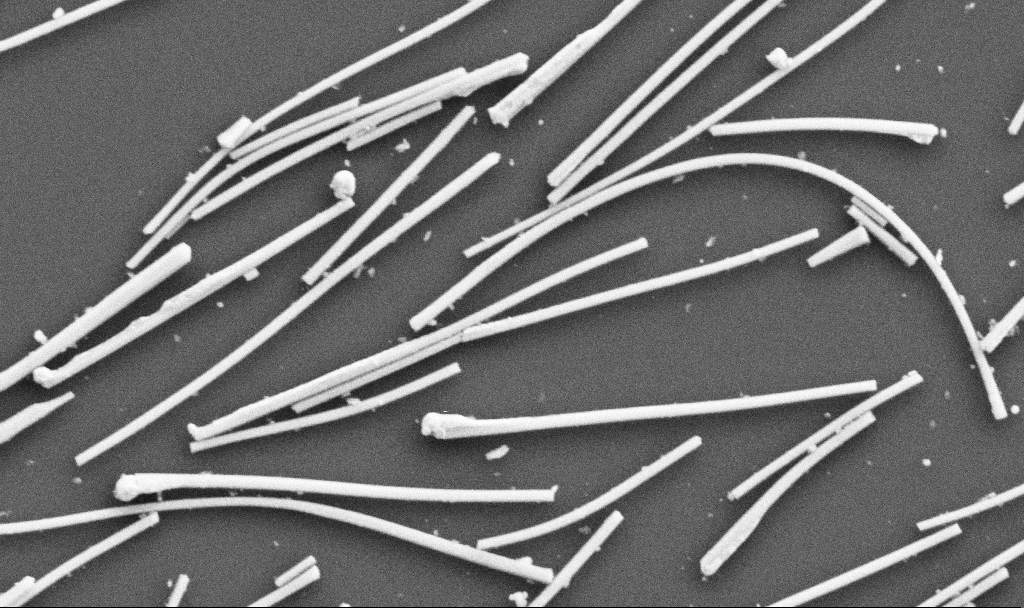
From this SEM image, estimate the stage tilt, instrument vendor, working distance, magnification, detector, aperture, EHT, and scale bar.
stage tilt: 0°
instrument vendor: Zeiss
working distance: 10.7 mm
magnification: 37.92 K X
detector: SE2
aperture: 30 µm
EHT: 5 kV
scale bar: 1000 nm